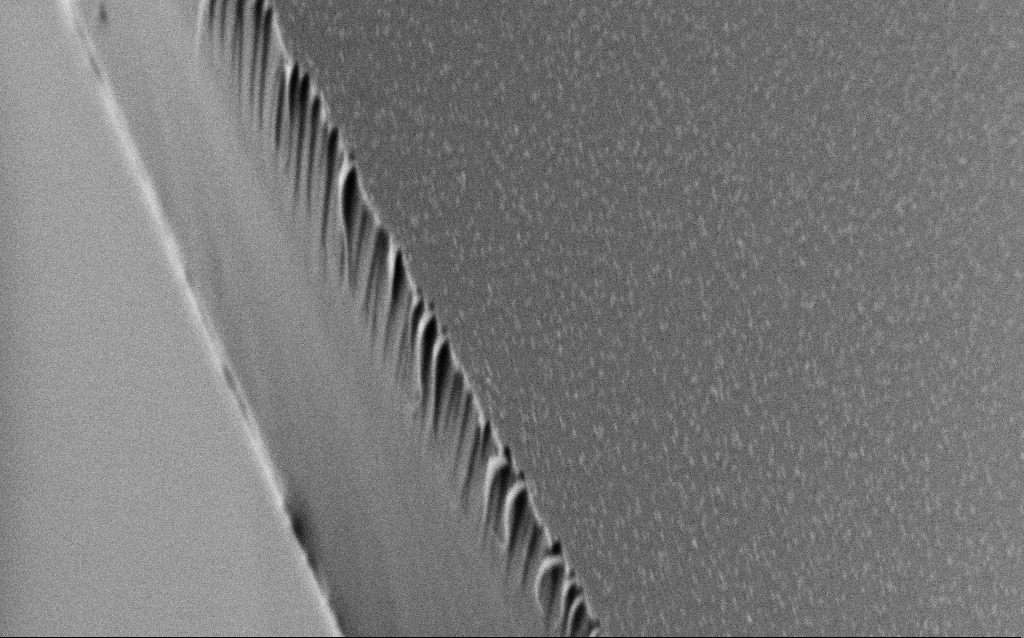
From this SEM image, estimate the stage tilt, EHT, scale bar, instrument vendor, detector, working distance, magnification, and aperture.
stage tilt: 44.9°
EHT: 2 kV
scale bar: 1000 nm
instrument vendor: Zeiss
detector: SE2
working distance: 6.9 mm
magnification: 50 K X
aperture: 30 µm